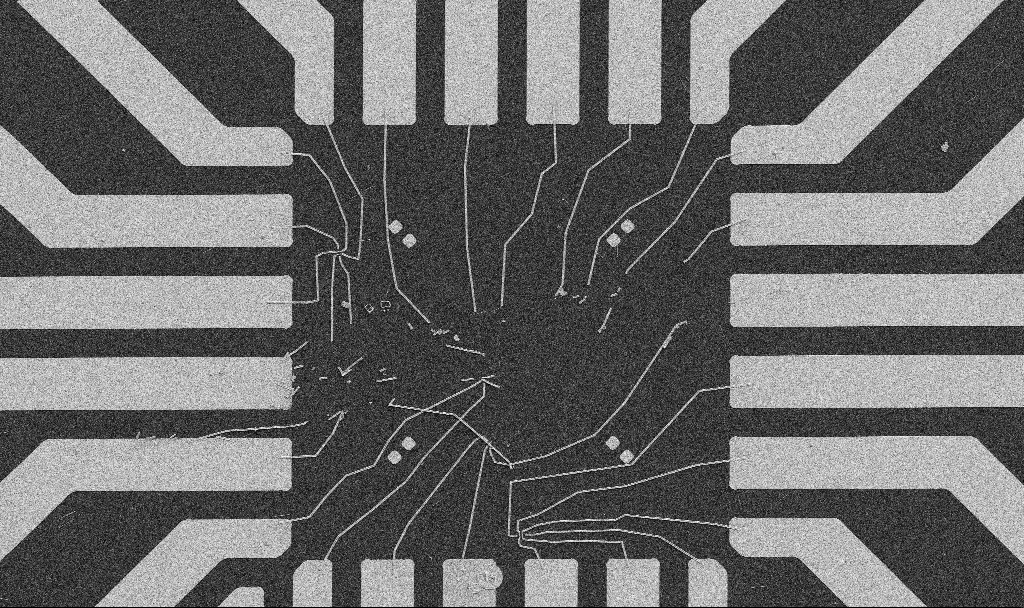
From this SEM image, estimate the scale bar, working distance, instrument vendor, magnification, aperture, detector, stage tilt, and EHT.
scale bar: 20000 nm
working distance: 10.7 mm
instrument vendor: Zeiss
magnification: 1 K X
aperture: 30 µm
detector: SE2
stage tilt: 0°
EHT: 5 kV